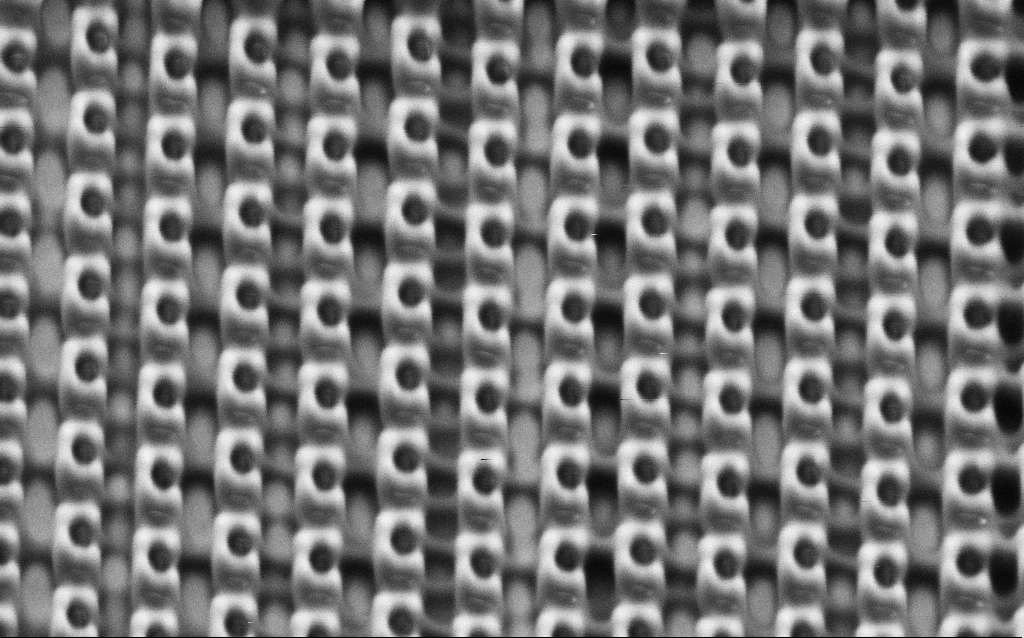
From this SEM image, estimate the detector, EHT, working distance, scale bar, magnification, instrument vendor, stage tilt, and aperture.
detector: SE2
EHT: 1.5 kV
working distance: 7.3 mm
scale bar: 1000 nm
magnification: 63.13 K X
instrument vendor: Zeiss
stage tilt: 30°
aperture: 30 µm